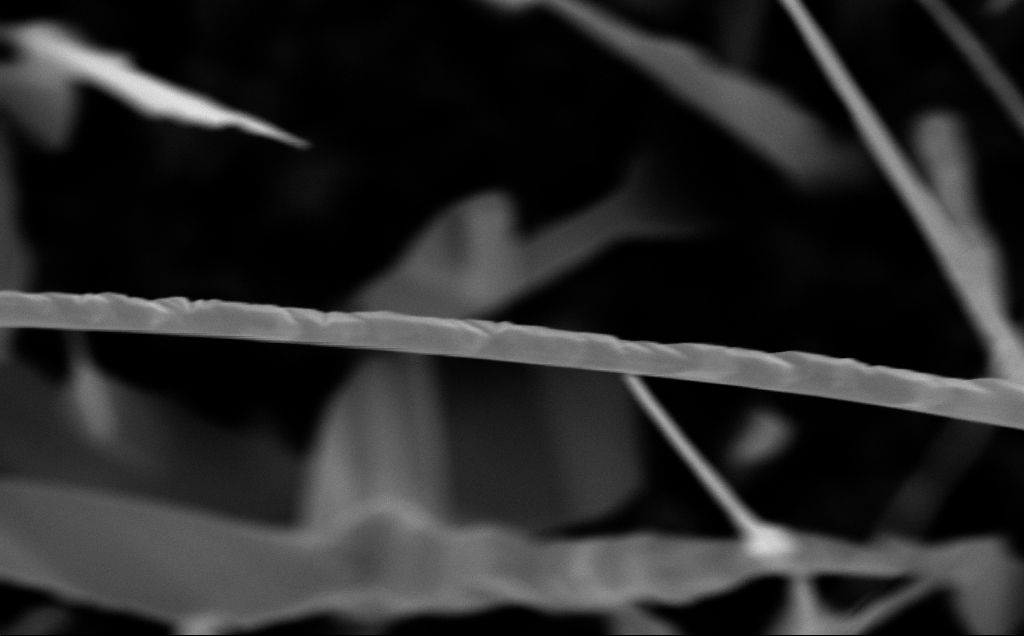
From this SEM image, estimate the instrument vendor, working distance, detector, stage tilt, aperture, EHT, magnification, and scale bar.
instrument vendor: Zeiss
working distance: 6 mm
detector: InLens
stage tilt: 0°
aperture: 30 µm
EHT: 10 kV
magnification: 100 K X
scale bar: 200 nm